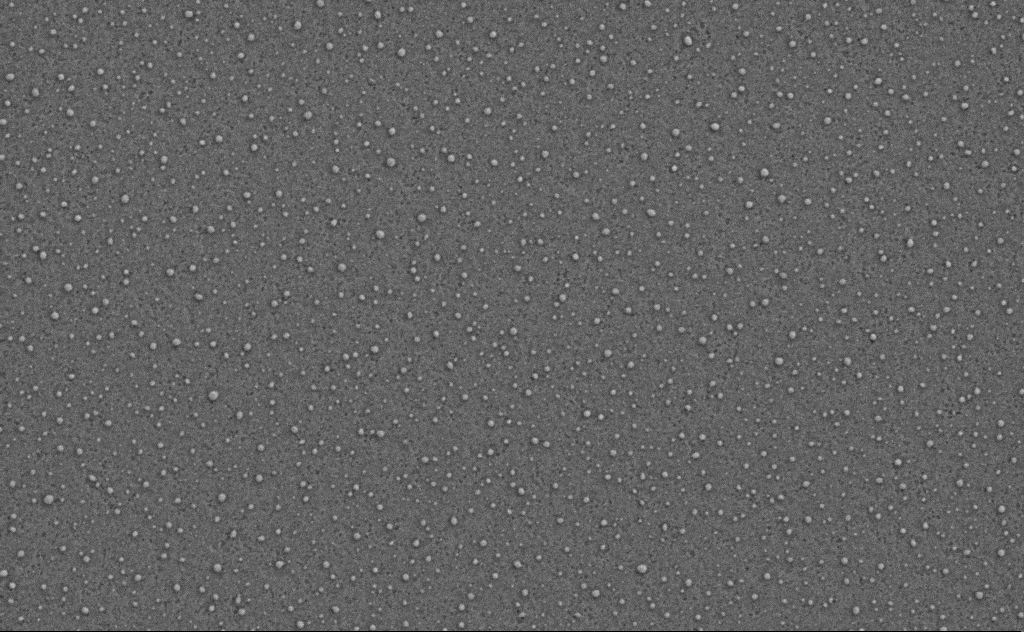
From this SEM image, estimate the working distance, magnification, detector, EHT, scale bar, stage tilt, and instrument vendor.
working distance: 4 mm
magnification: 40 K X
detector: SE2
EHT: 3 kV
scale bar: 1000 nm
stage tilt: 0°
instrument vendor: Zeiss